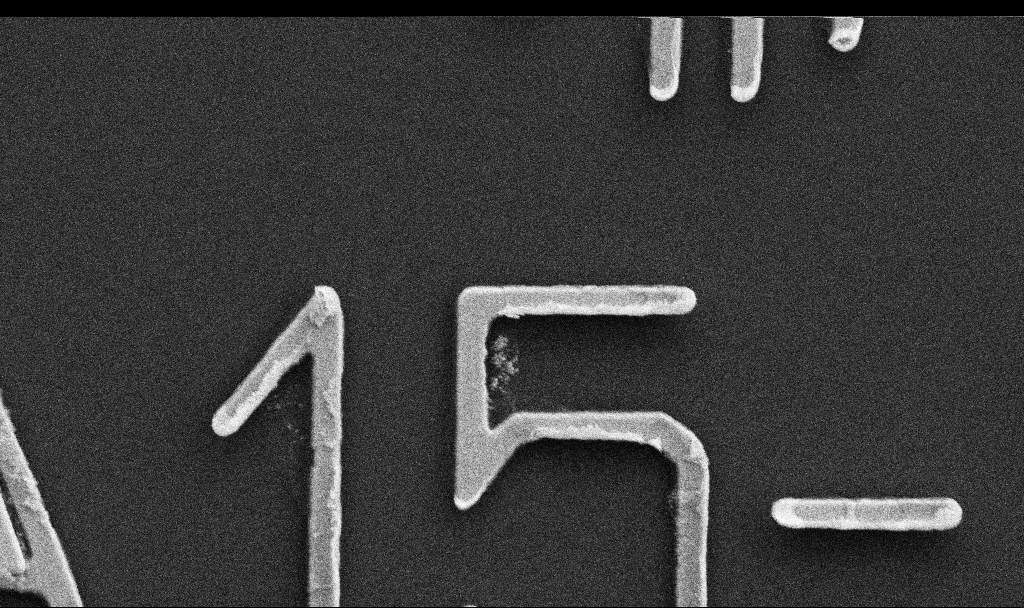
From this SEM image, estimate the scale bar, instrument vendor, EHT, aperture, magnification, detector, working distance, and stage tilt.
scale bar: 1000 nm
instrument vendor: Zeiss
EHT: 5 kV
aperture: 30 µm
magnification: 36.63 K X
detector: SE2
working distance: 10.7 mm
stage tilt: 0°